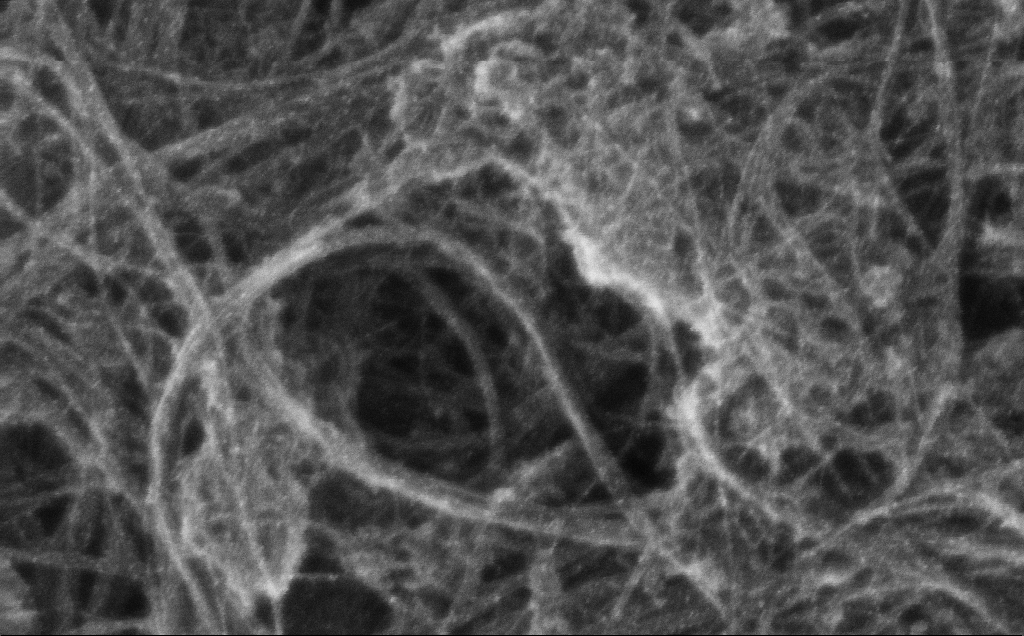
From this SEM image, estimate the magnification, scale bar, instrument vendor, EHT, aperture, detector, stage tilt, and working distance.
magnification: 234.09 K X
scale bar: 200 nm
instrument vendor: Zeiss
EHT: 10 kV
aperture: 30 µm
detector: InLens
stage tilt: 0°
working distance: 3 mm